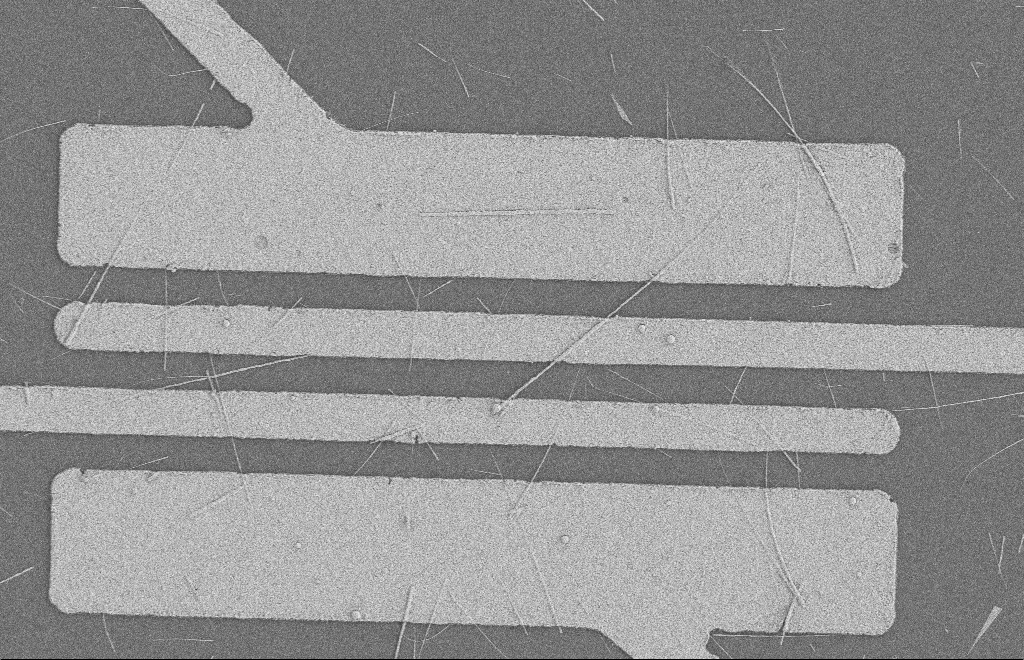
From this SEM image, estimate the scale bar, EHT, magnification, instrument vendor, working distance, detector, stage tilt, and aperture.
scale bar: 2000 nm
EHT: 2 kV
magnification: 5.1 K X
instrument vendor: Zeiss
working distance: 8 mm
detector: SE2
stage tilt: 0°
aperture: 20 µm